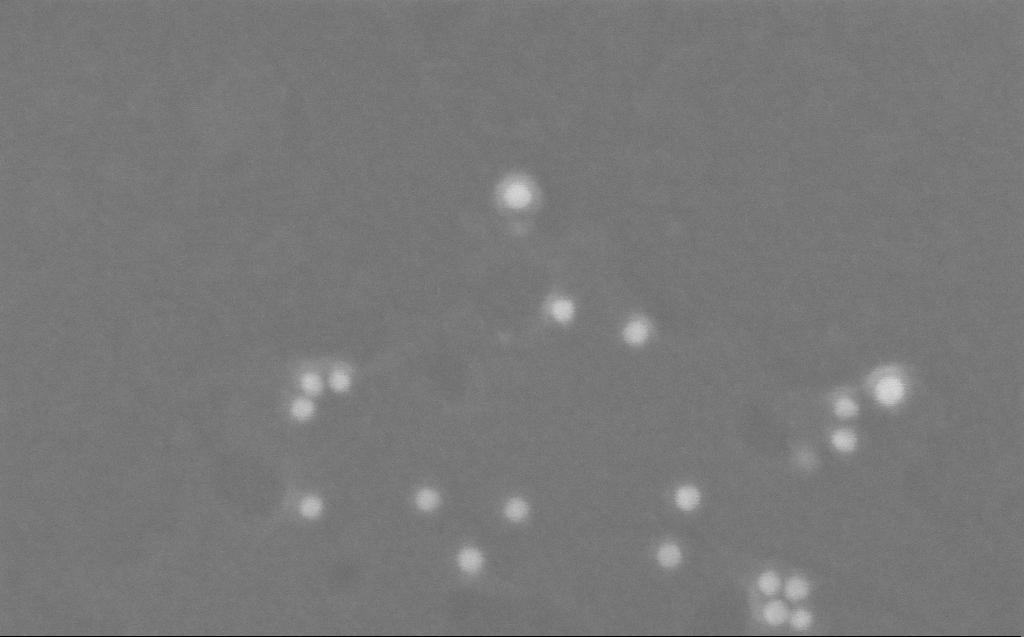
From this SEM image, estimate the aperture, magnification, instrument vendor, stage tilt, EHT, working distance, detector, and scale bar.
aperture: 30 µm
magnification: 544.84 K X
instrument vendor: Zeiss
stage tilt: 0°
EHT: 10 kV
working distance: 3 mm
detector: InLens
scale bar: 100 nm